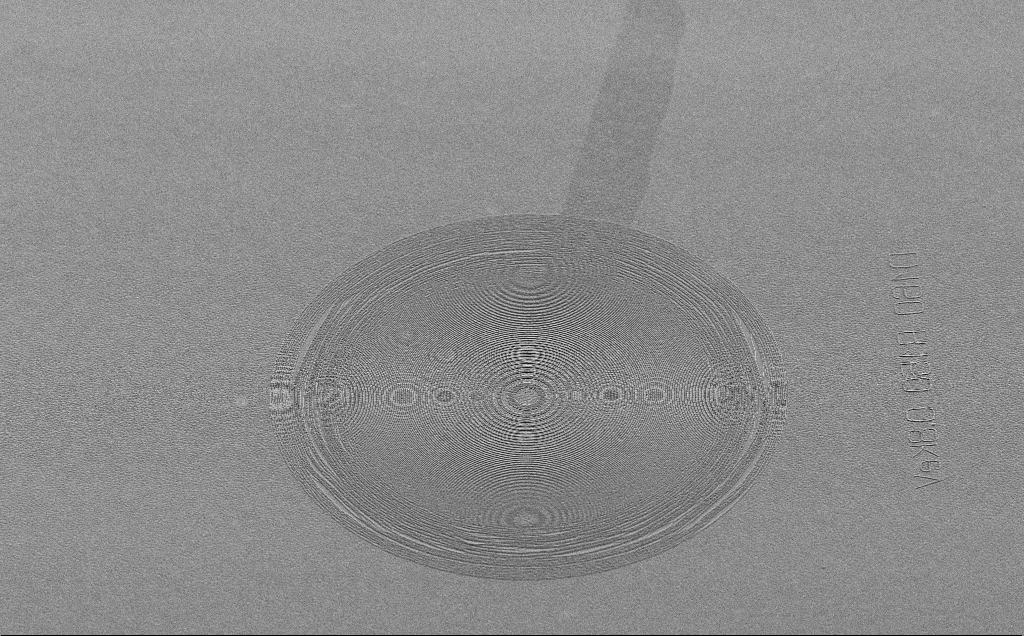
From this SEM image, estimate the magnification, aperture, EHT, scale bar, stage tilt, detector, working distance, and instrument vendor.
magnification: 1.2 K X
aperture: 30 µm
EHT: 5 kV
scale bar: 20000 nm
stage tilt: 45°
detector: SE2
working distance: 6 mm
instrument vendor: Zeiss